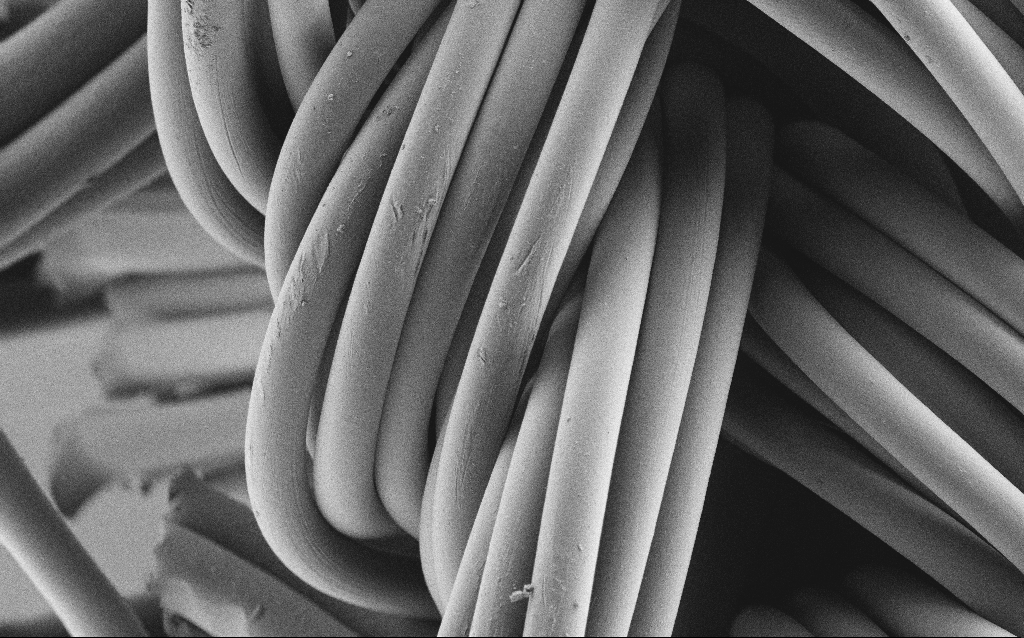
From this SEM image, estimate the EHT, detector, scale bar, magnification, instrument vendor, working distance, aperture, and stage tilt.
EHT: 1 kV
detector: SE2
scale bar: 20000 nm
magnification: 0.927 K X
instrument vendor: Zeiss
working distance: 4 mm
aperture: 30 µm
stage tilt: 0°